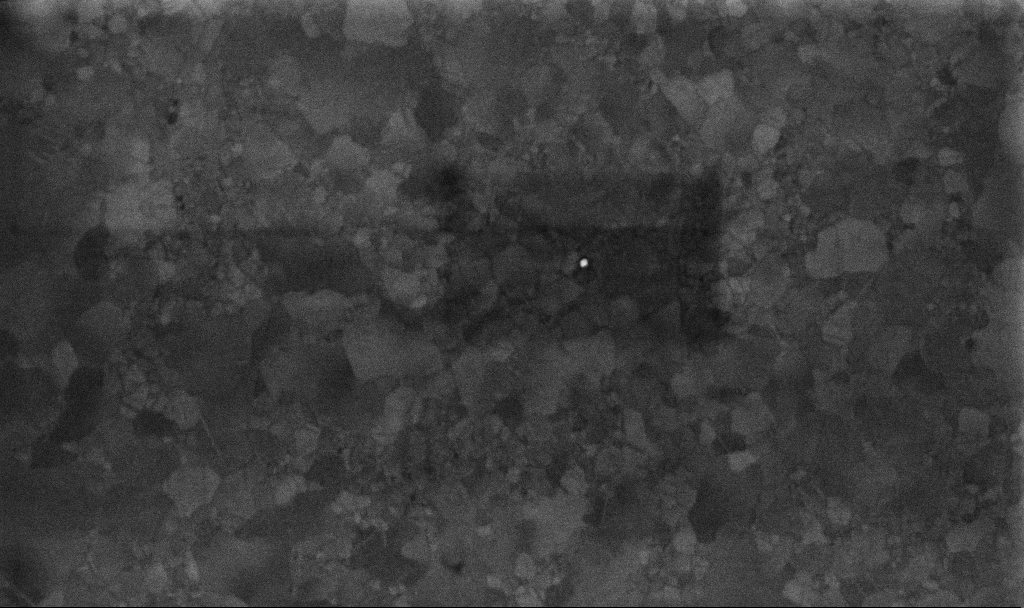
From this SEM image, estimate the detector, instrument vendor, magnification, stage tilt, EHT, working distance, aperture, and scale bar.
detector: InLens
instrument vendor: Zeiss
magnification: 100 K X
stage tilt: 0°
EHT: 10 kV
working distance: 3.4 mm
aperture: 30 µm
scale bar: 200 nm